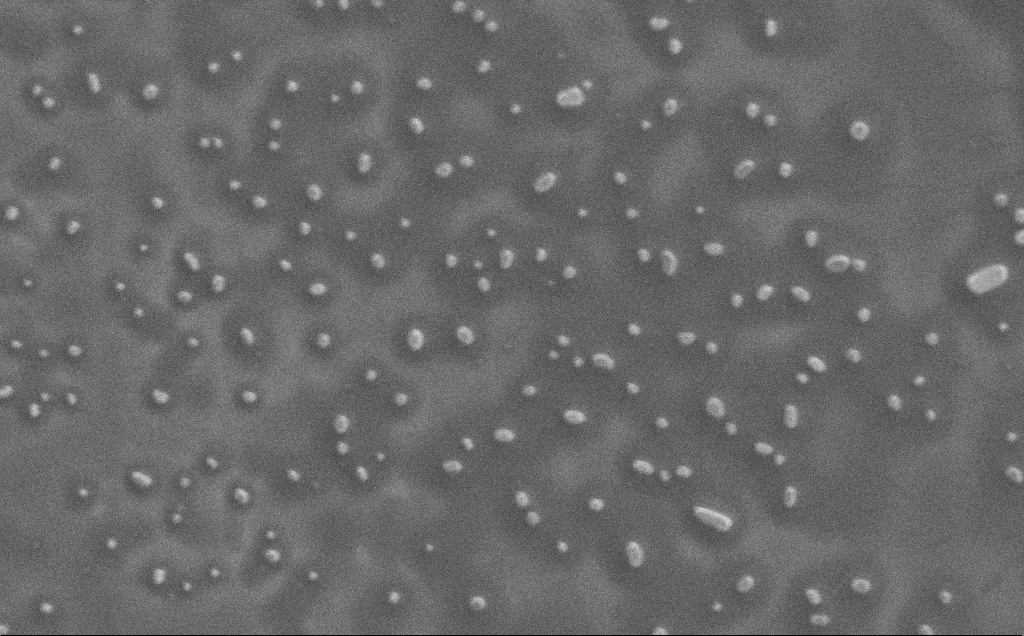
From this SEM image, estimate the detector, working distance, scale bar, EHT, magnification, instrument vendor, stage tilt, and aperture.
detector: SE2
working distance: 3 mm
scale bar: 2000 nm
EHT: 1 kV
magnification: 7.6 K X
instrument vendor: Zeiss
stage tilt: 0°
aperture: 30 µm